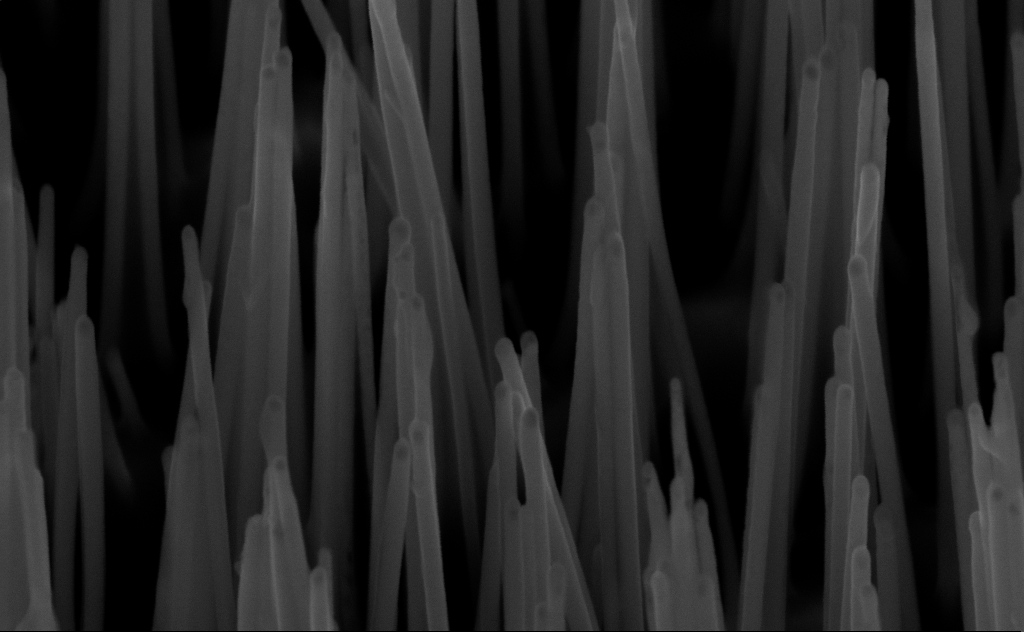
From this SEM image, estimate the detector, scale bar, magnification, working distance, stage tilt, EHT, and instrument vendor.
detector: InLens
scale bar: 100 nm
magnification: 150 K X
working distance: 6 mm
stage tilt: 45°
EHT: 10 kV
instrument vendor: Zeiss